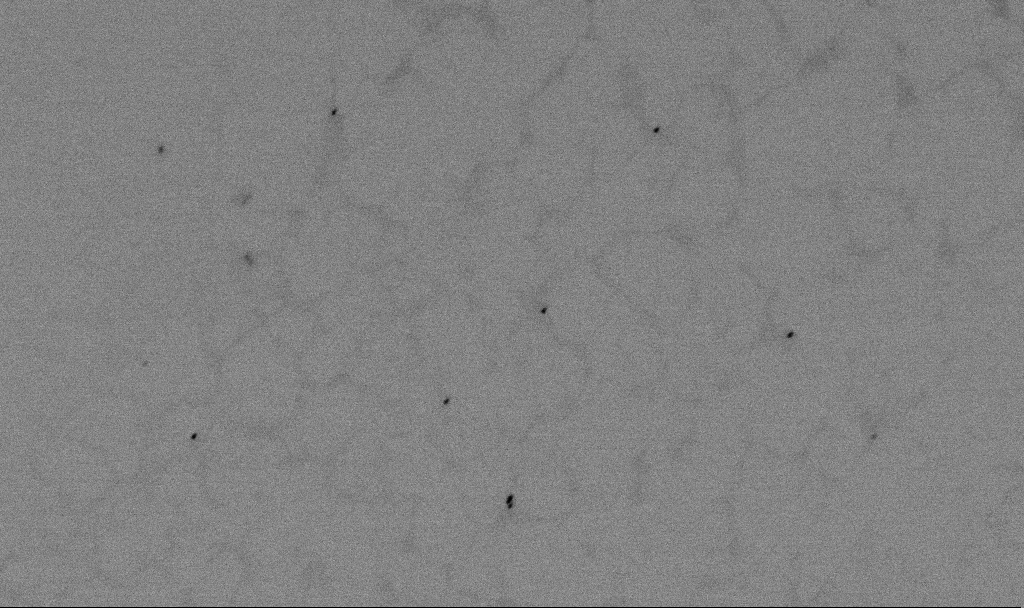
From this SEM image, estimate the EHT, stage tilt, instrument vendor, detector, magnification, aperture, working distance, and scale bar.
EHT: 2 kV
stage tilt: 0°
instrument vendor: Zeiss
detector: SE2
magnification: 60 K X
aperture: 30 µm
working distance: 5.5 mm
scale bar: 1000 nm